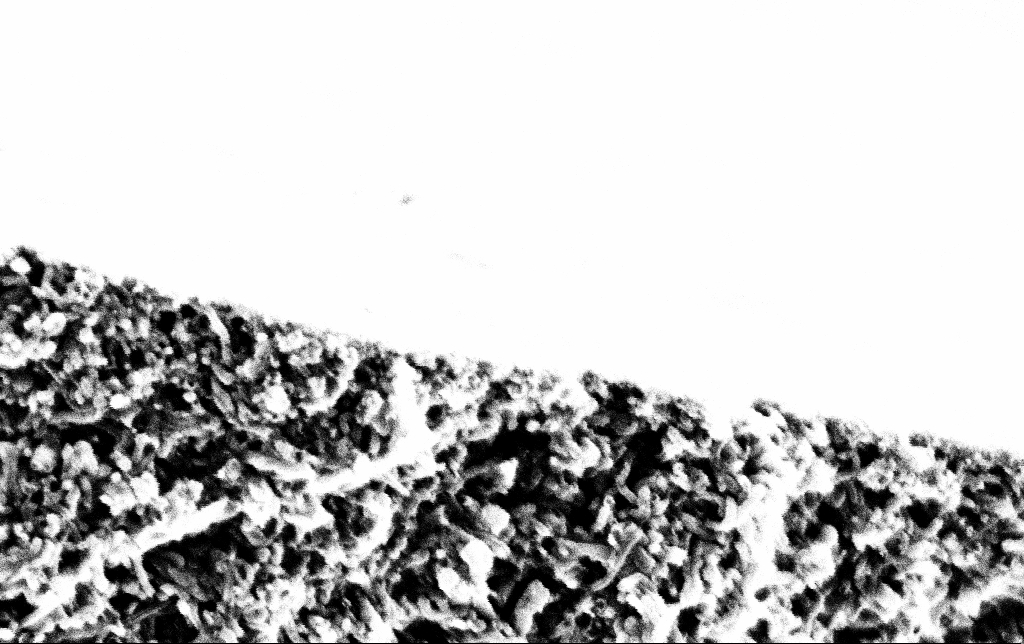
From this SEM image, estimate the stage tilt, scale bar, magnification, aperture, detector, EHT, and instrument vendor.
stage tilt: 0°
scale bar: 200 nm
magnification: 100 K X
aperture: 30 µm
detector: InLens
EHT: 2 kV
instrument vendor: Zeiss